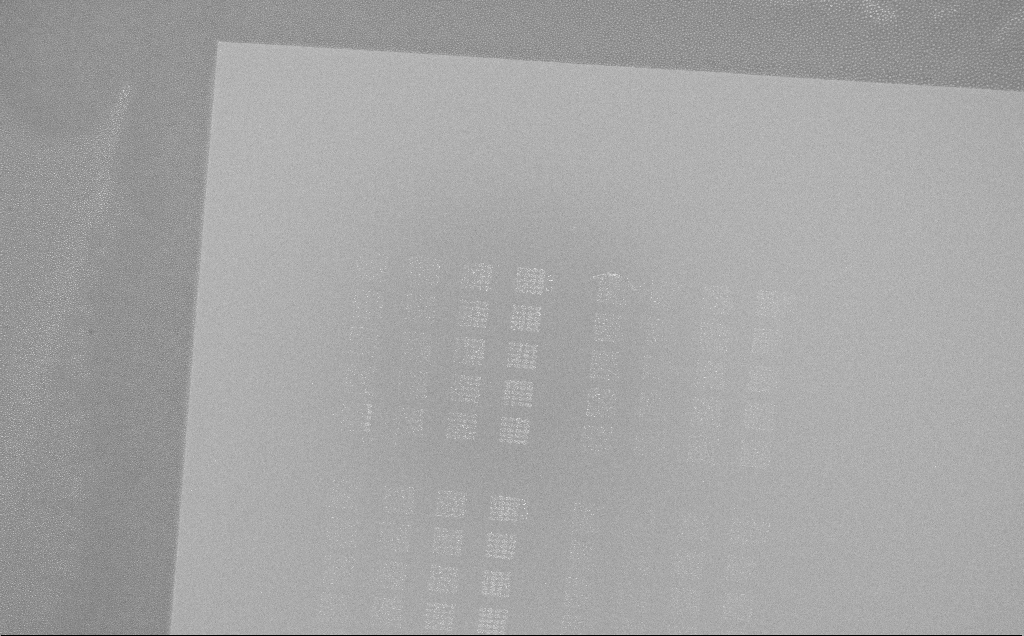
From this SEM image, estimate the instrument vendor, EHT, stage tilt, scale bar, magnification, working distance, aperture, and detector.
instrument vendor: Zeiss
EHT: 5 kV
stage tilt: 0°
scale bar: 100000 nm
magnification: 0.281 K X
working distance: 6 mm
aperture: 30 µm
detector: InLens